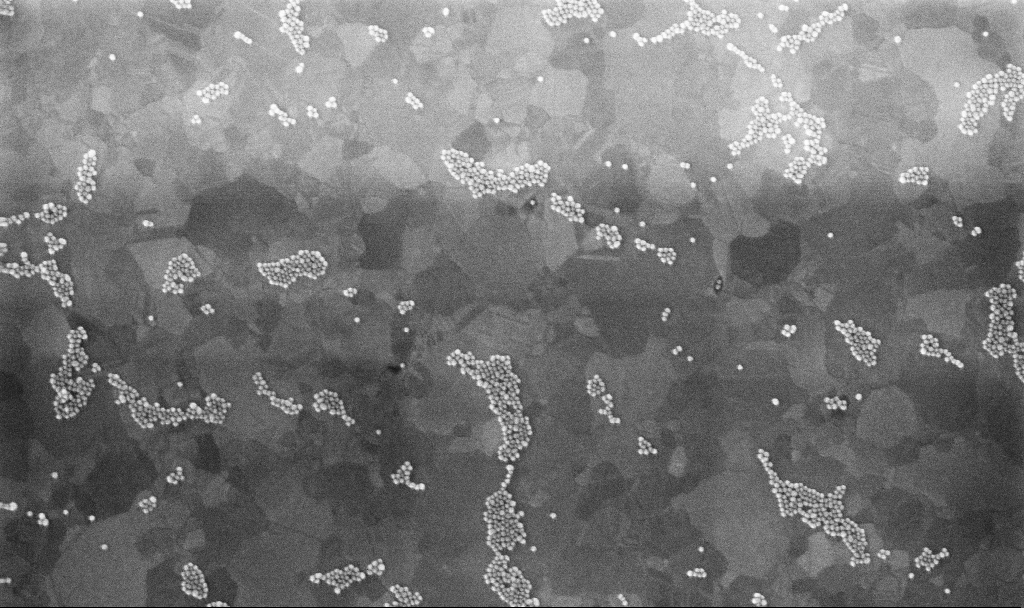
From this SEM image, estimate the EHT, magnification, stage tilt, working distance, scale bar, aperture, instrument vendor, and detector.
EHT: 10 kV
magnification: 100 K X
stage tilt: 0°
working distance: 3.4 mm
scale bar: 200 nm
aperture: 30 µm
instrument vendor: Zeiss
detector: InLens